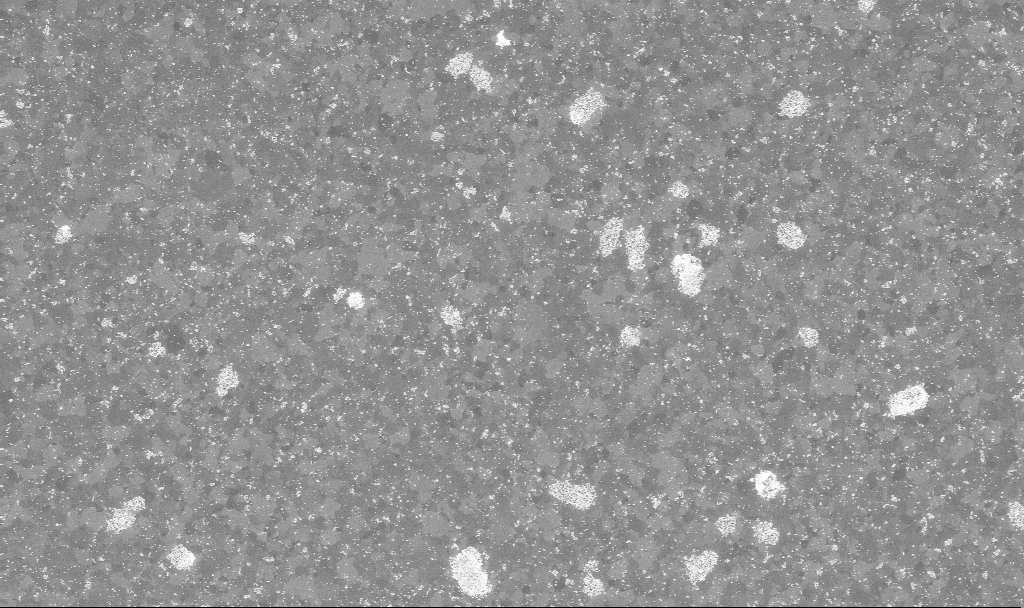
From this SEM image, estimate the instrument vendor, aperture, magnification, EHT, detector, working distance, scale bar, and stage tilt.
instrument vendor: Zeiss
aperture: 30 µm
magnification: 20 K X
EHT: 10 kV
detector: InLens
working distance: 3.8 mm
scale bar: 1000 nm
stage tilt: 0°